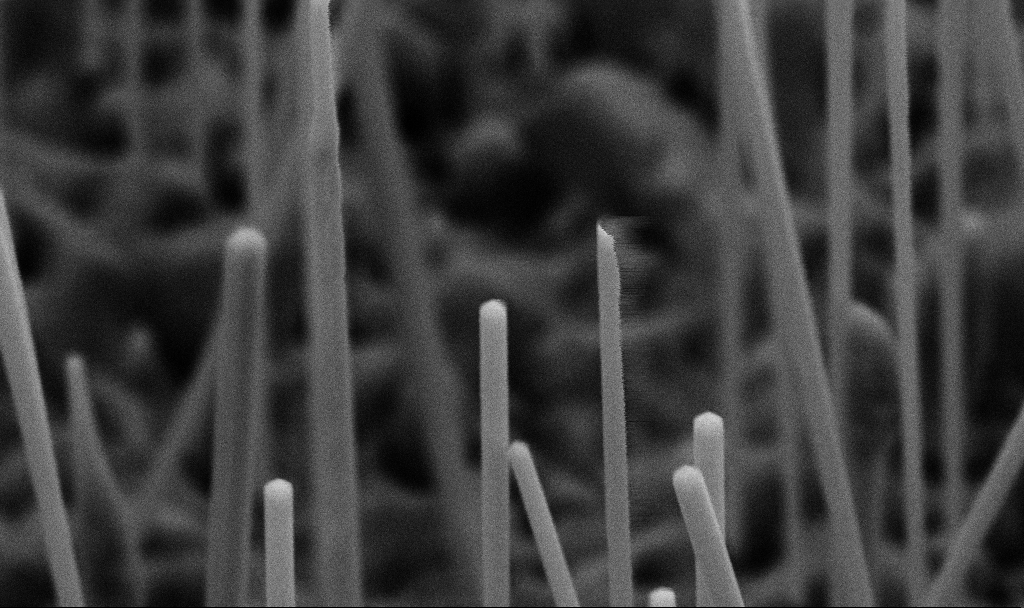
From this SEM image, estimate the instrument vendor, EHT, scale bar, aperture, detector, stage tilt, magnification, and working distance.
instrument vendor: Zeiss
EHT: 5 kV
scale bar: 200 nm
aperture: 30 µm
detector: SE2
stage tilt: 45°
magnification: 82.25 K X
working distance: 7.2 mm